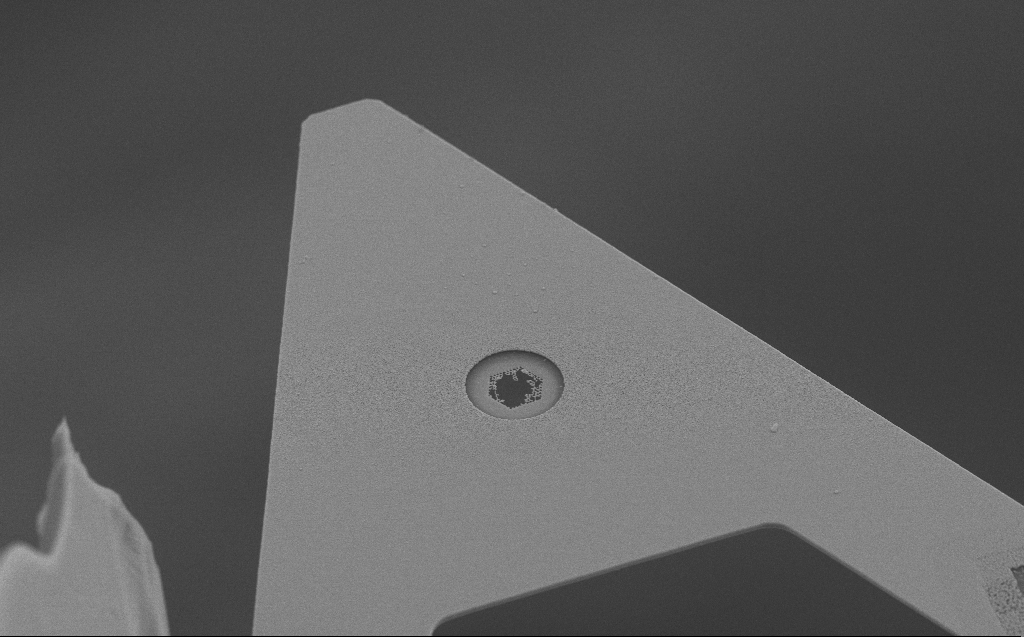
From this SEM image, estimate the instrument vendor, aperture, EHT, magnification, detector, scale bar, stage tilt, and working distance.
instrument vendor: Zeiss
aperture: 30 µm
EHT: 10 kV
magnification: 5.16 K X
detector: SE2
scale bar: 10000 nm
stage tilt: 45°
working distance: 6 mm